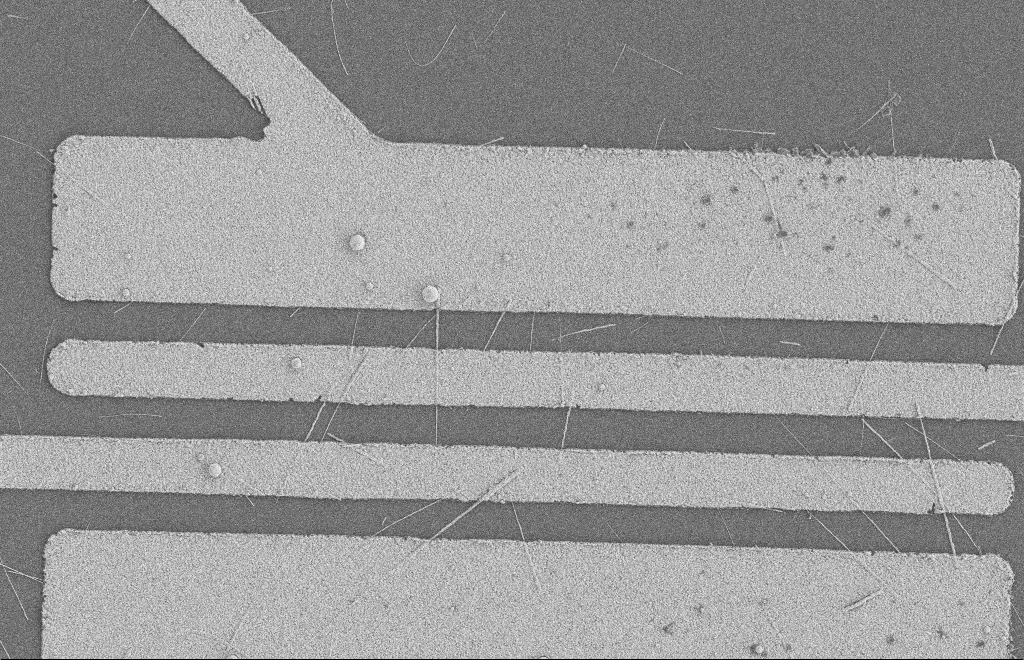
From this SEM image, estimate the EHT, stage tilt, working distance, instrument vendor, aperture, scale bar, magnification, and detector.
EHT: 2 kV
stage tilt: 0°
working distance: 8 mm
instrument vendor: Zeiss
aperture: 20 µm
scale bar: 2000 nm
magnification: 5.81 K X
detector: SE2